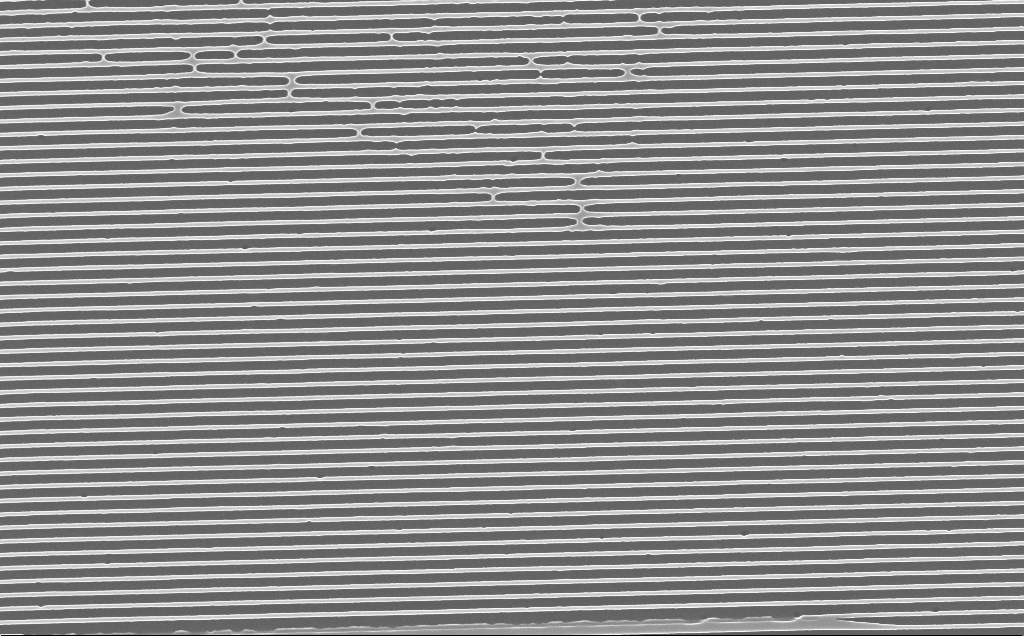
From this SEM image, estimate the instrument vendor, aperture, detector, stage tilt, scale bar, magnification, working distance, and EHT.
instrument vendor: Zeiss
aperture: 30 µm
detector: InLens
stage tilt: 0°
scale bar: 2000 nm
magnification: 20.01 K X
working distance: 4 mm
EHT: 10 kV